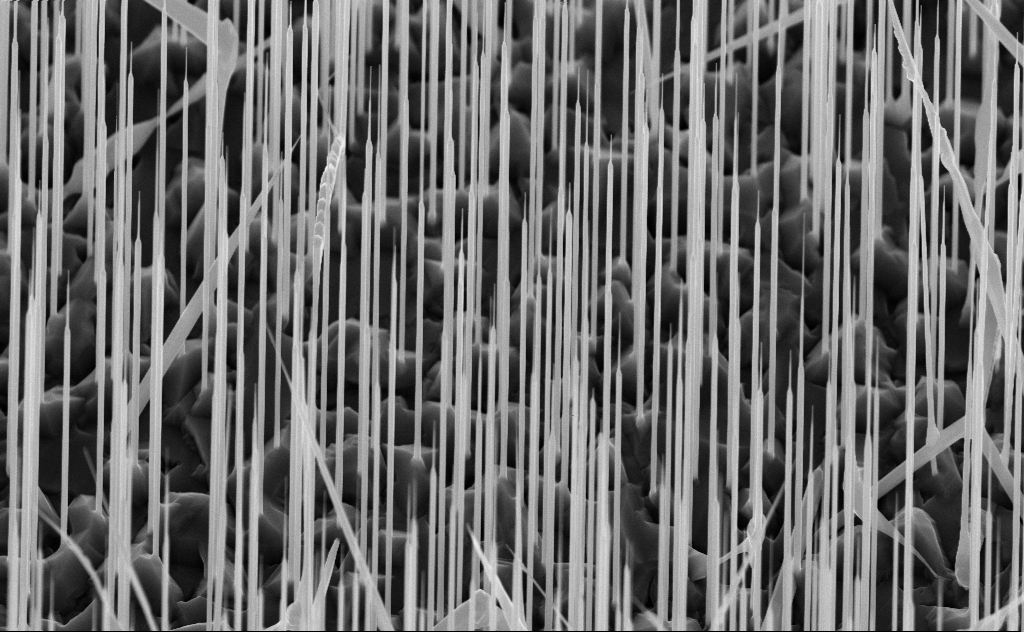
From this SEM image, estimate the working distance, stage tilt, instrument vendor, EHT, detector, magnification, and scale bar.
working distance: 6 mm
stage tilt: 45°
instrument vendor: Zeiss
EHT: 10 kV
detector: InLens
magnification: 20 K X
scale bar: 2000 nm